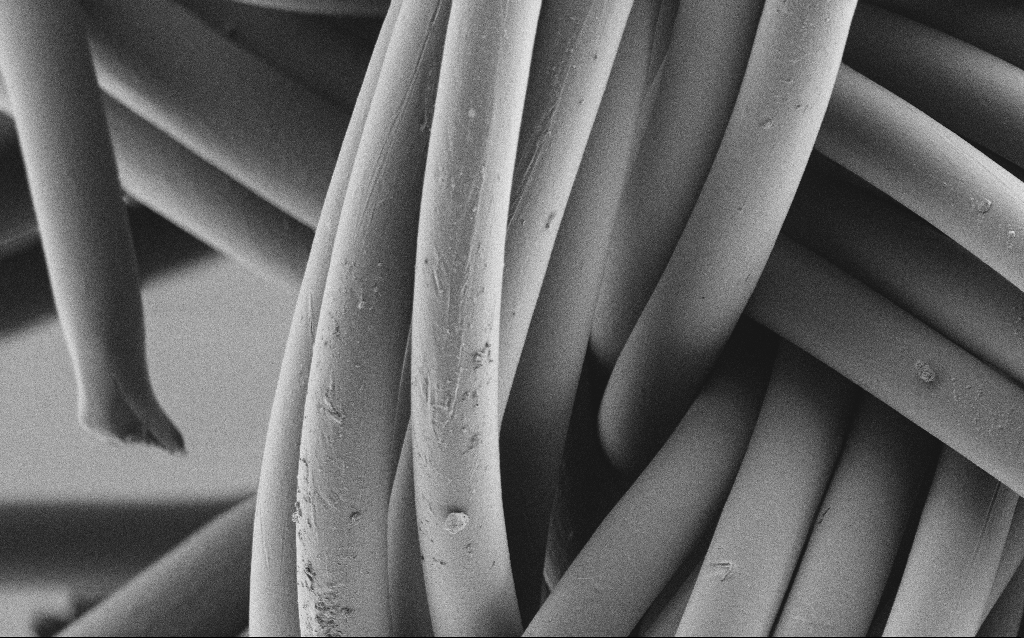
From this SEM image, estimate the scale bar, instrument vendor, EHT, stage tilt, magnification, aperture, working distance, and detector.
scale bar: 20000 nm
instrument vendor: Zeiss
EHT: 1 kV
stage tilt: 0°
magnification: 1.36 K X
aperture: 30 µm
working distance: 4 mm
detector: SE2